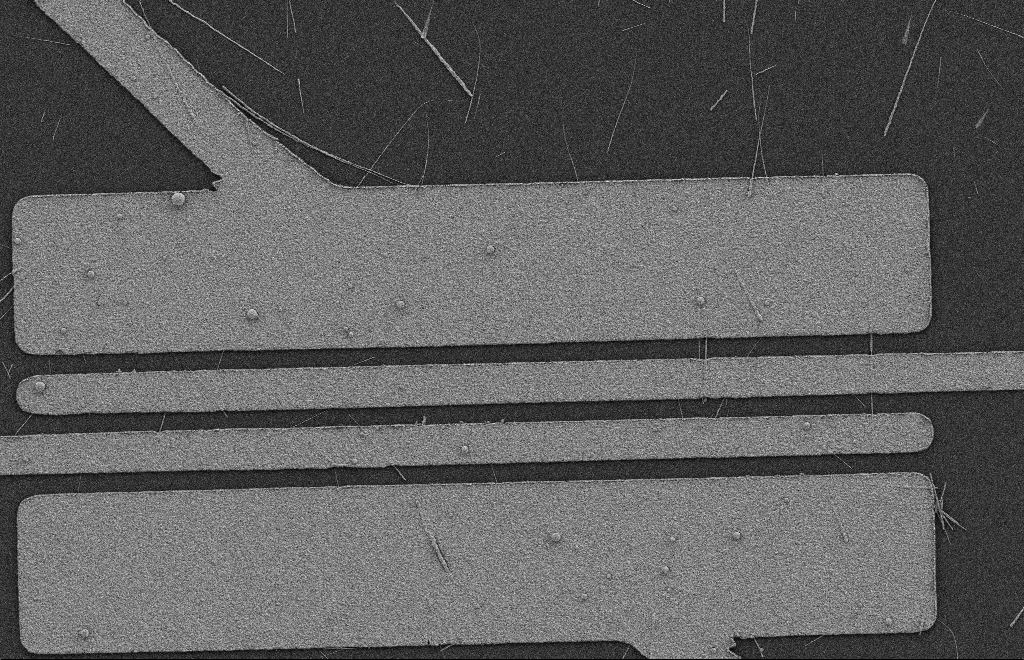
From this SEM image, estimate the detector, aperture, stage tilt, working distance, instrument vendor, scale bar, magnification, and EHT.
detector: SE2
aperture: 20 µm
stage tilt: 0°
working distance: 9 mm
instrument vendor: Zeiss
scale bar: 2000 nm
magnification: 5.5 K X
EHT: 2 kV